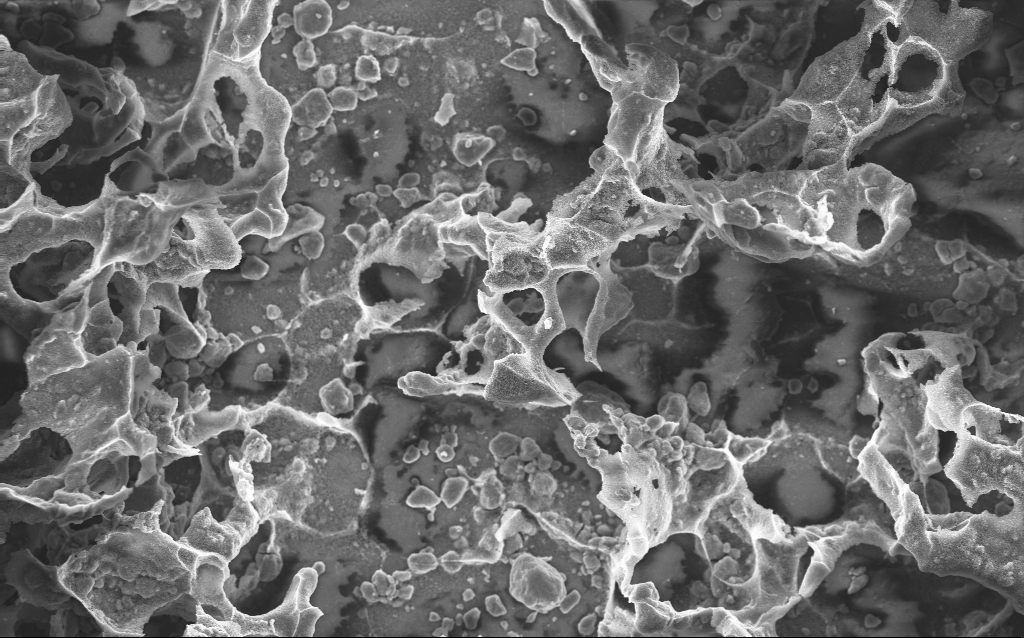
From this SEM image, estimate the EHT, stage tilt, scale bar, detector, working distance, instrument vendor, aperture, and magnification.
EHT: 10 kV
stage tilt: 0°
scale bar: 10000 nm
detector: InLens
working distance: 2.7 mm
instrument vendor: Zeiss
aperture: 30 µm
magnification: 1.69 K X